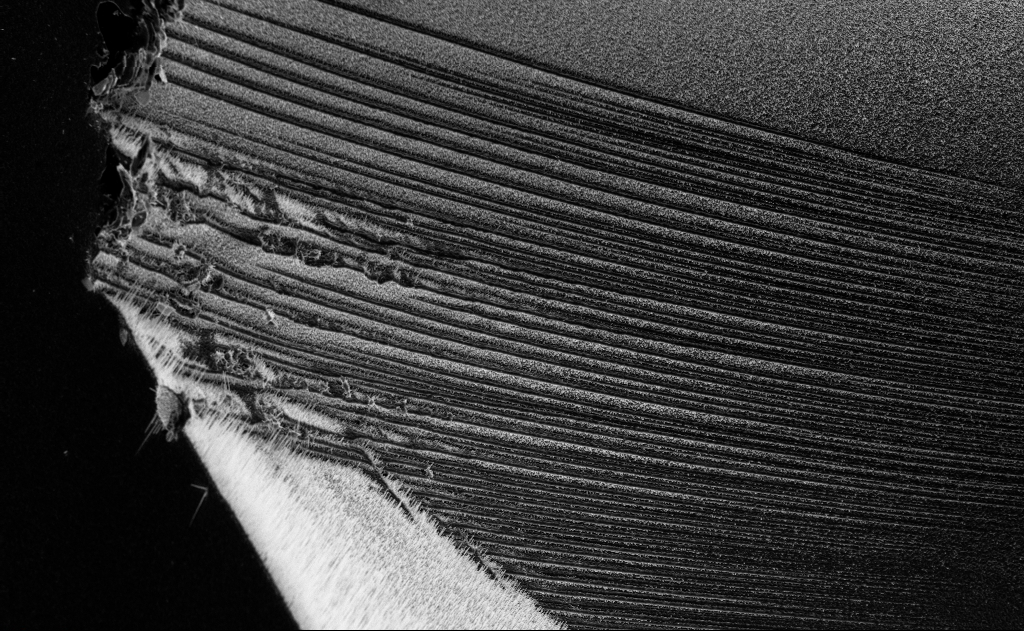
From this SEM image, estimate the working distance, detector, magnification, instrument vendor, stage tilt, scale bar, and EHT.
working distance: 6 mm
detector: InLens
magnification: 1.38 K X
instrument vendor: Zeiss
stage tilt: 0°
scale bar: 10000 nm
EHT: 10 kV